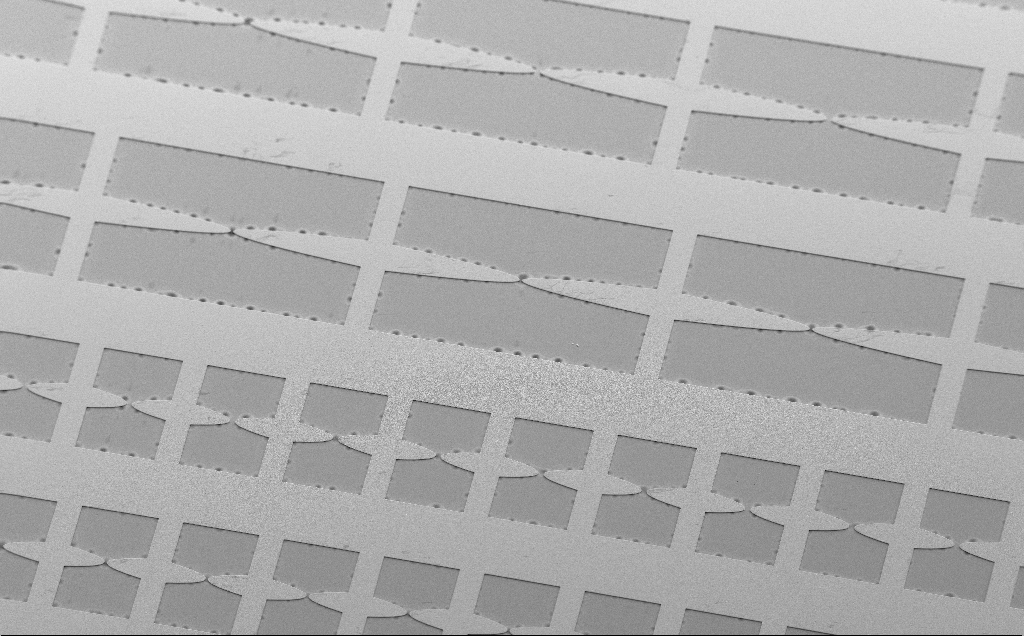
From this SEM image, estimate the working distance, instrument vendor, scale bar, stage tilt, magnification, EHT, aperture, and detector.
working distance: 13 mm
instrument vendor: Zeiss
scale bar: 200000 nm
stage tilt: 35°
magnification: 0.185 K X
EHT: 5 kV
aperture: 30 µm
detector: SE2